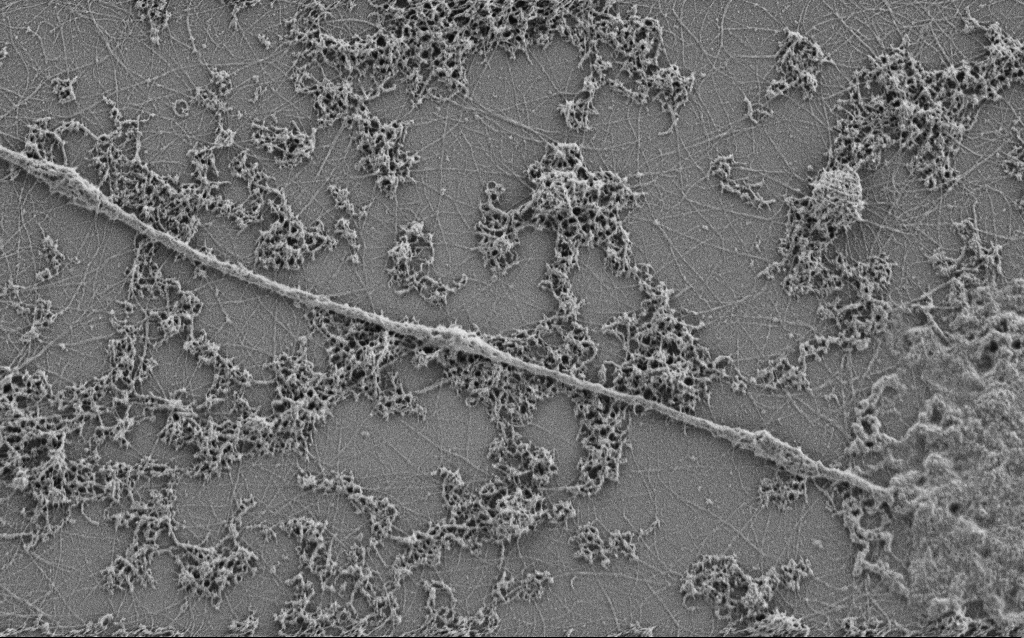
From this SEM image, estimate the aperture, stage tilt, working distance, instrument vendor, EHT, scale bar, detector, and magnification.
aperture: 30 µm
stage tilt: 0°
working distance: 4 mm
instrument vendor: Zeiss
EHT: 0.9 kV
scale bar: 1000 nm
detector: SE2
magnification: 15 K X